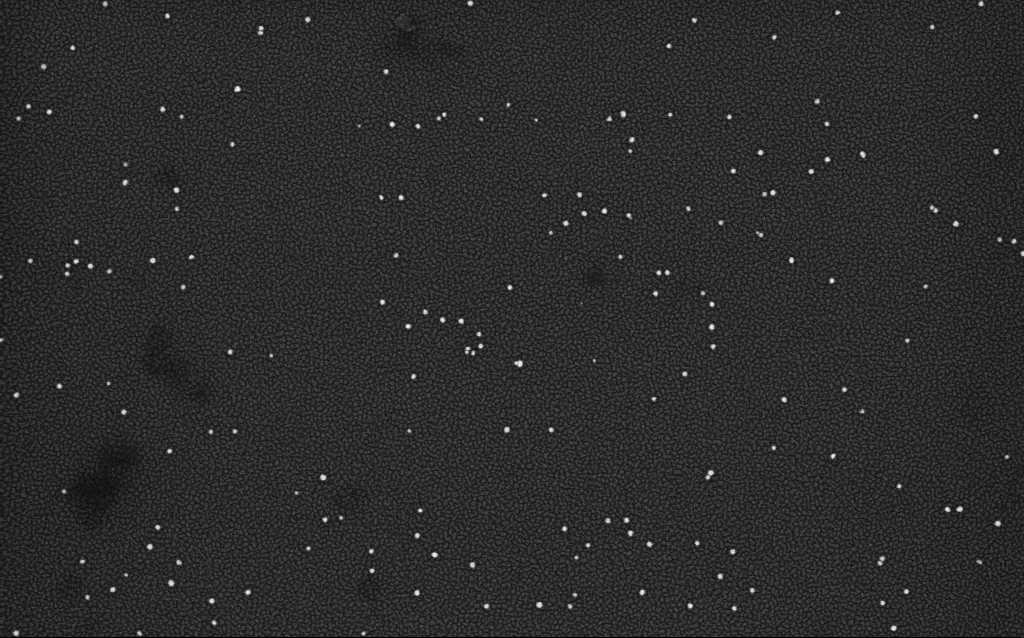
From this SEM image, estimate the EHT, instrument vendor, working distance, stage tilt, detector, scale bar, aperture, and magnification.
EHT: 4 kV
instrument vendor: Zeiss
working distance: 2.1 mm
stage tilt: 0°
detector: InLens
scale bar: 200 nm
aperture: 30 µm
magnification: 100 K X